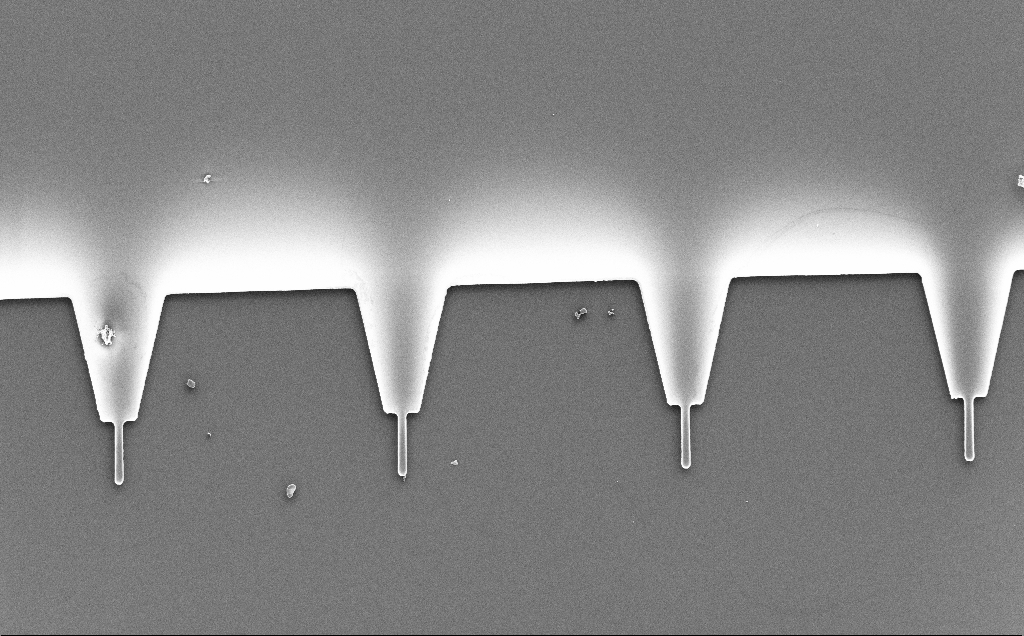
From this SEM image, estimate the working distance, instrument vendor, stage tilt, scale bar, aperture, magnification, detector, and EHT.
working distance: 7 mm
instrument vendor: Zeiss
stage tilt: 0°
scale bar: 100000 nm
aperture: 30 µm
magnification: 0.656 K X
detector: SE2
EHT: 5 kV